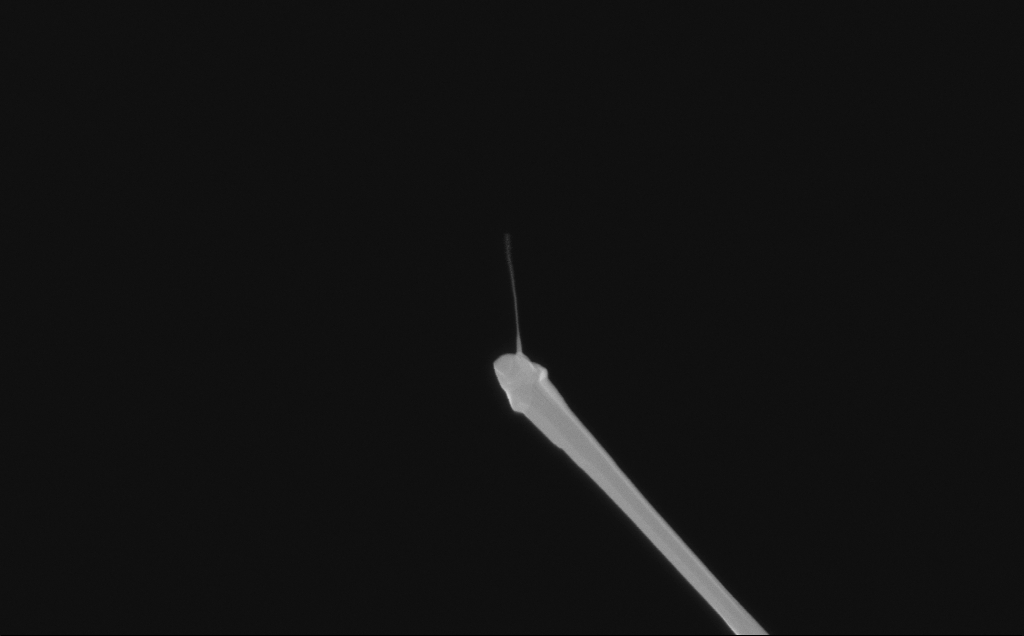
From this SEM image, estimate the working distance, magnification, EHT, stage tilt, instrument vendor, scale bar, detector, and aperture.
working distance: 6 mm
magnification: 81.98 K X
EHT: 10 kV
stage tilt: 0°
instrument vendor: Zeiss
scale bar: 200 nm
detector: InLens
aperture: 30 µm